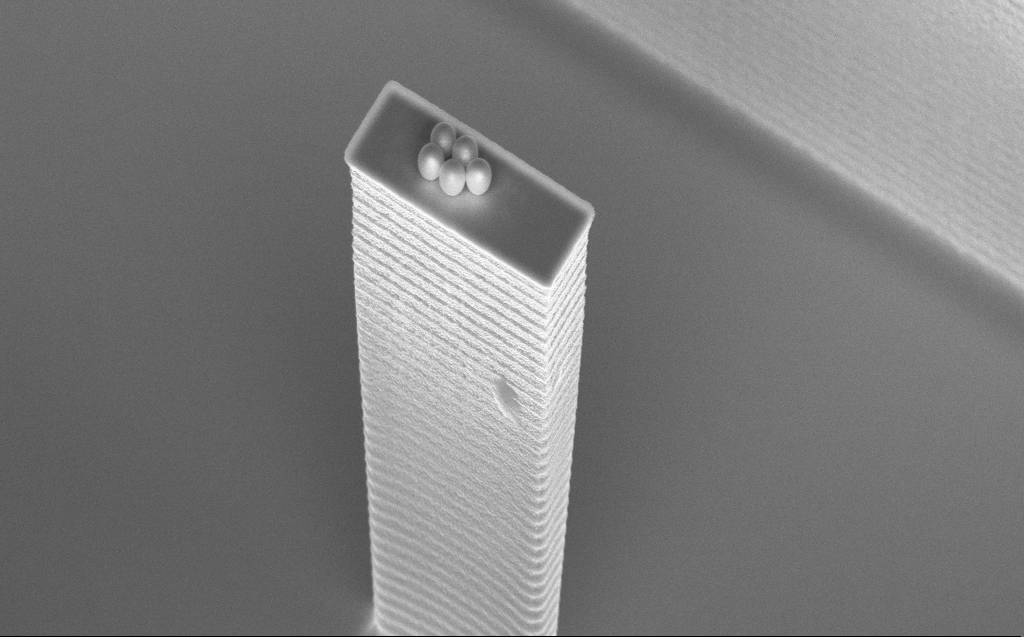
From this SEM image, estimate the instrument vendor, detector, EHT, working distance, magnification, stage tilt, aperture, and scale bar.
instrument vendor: Zeiss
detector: InLens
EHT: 5 kV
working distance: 7 mm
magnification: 11.89 K X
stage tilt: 45°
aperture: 30 µm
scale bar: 2000 nm